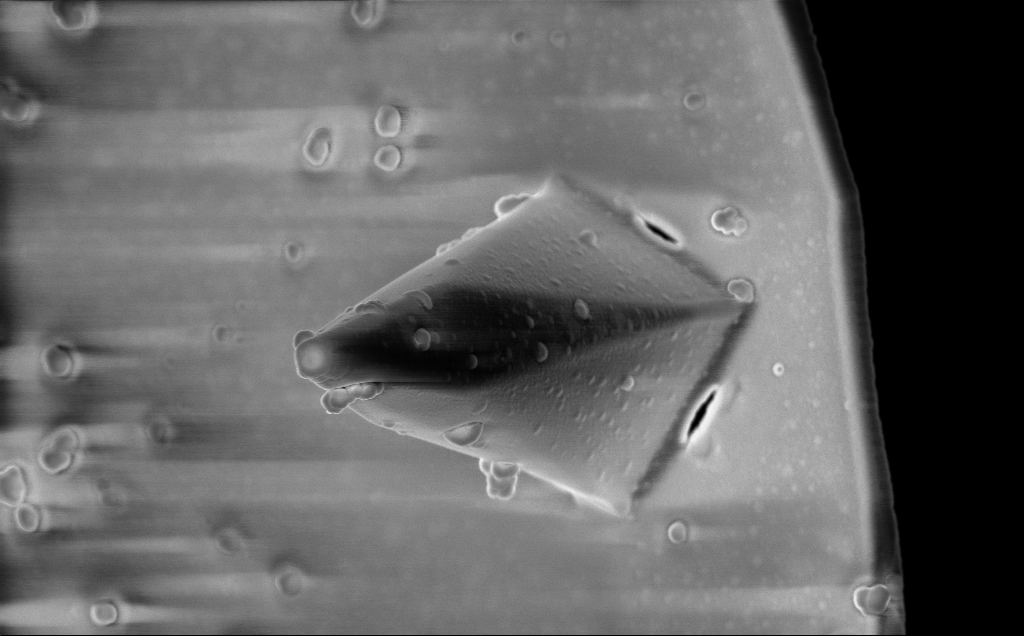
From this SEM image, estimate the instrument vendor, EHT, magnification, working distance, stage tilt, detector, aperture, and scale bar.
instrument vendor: Zeiss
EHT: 5 kV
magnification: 15.29 K X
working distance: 8 mm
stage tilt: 0°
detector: InLens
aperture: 30 µm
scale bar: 2000 nm